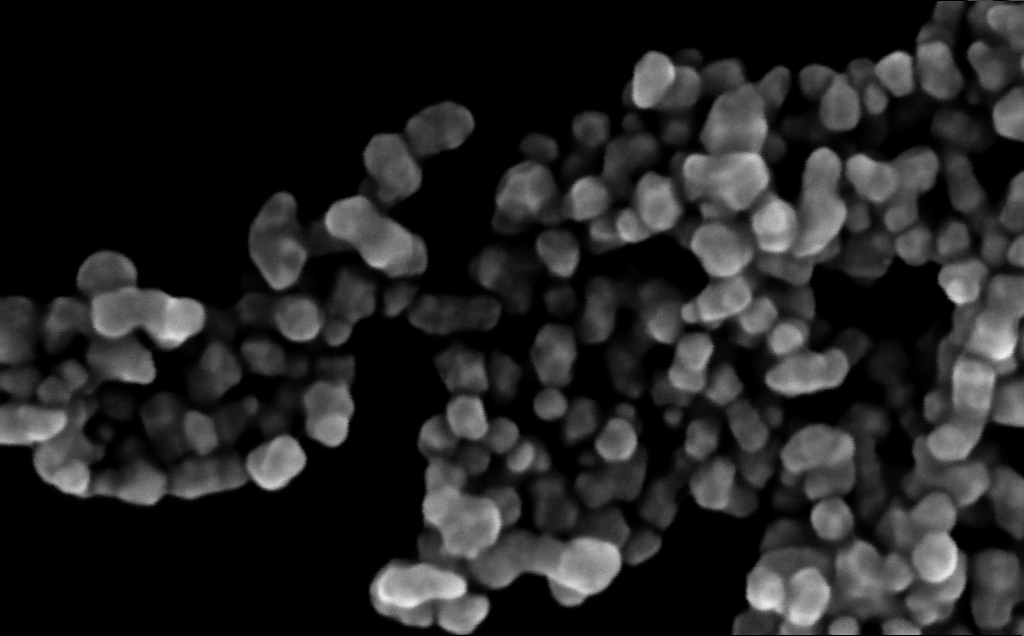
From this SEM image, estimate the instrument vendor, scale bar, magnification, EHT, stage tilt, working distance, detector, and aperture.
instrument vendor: Zeiss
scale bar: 100 nm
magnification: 403.01 K X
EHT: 10 kV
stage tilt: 0°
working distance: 4 mm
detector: InLens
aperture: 30 µm